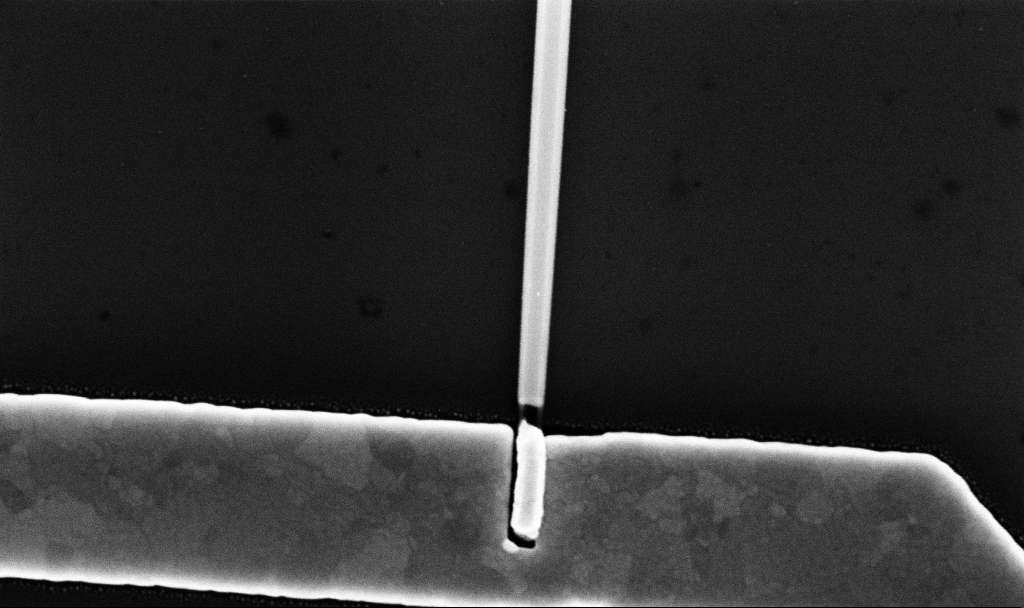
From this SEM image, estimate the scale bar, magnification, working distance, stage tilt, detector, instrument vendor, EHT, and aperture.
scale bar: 200 nm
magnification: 72.91 K X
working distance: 7.7 mm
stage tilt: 0°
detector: InLens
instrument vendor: Zeiss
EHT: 10 kV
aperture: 30 µm